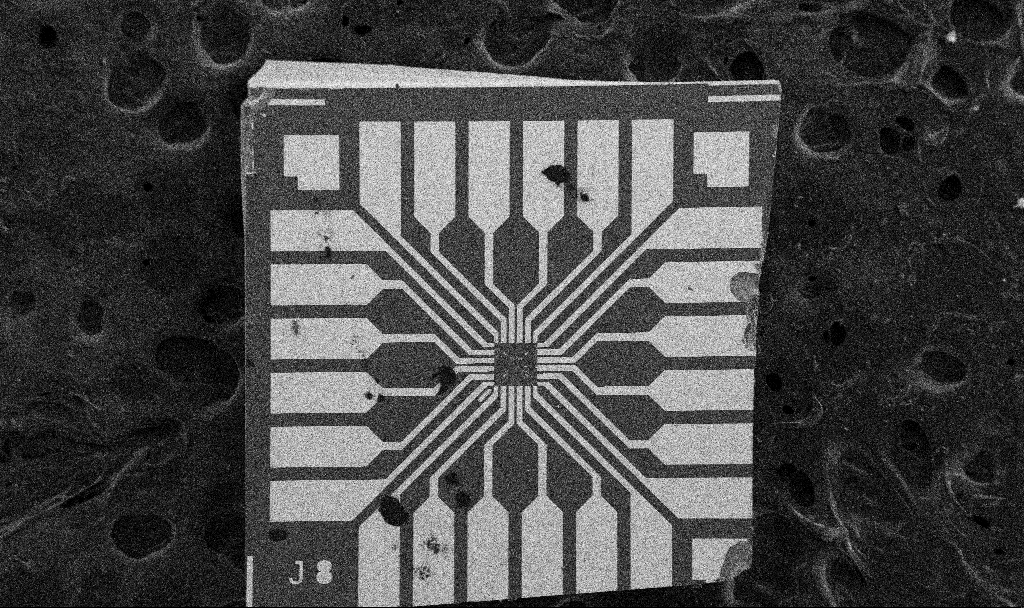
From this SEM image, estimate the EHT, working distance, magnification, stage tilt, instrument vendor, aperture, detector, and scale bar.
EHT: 5 kV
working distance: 8.7 mm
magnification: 0.1 K X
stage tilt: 0°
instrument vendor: Zeiss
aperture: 30 µm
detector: SE2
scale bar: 200000 nm